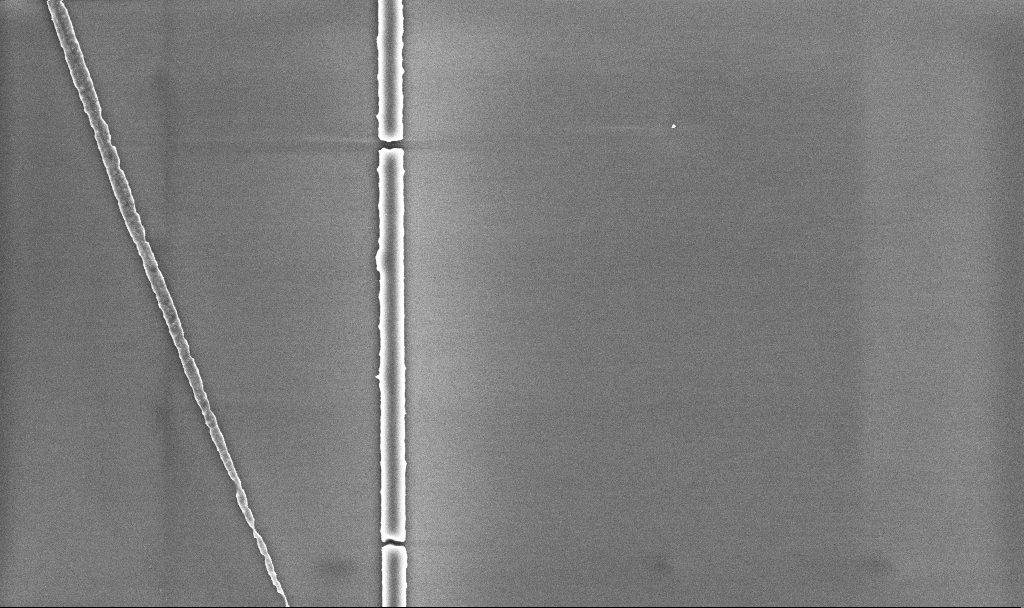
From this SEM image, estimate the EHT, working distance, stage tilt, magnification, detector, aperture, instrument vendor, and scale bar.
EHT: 5 kV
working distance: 5.2 mm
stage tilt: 0°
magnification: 17.99 K X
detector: InLens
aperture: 30 µm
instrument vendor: Zeiss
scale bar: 2000 nm